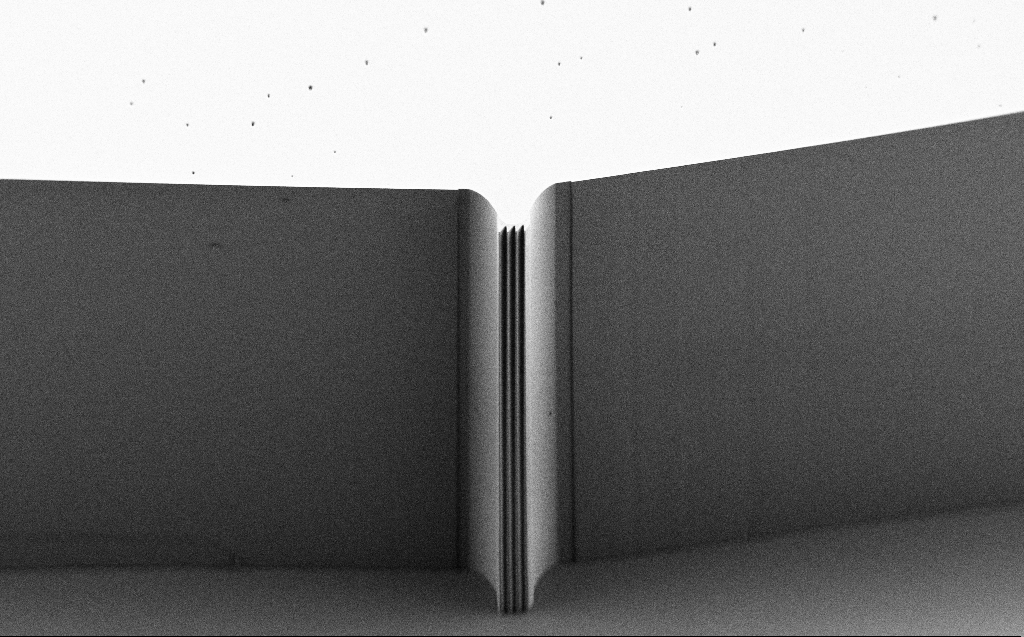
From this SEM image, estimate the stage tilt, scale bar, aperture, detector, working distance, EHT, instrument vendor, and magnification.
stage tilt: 45°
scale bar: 100000 nm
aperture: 30 µm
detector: SE2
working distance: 4 mm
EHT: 0.9 kV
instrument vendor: Zeiss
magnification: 0.288 K X